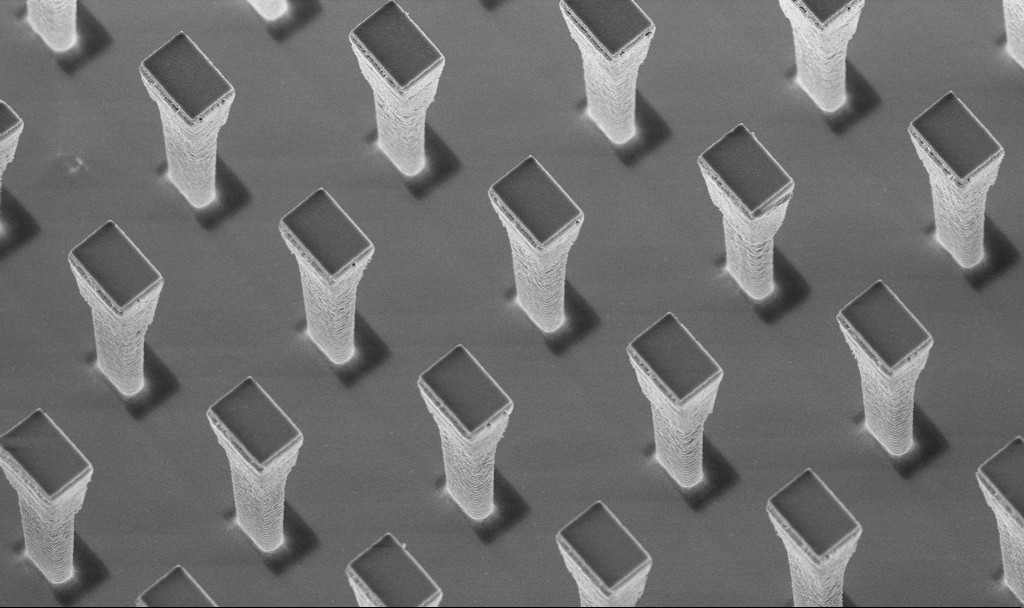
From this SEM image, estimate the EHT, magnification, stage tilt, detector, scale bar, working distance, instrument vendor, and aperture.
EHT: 5 kV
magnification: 6.66 K X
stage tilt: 20°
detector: InLens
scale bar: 10000 nm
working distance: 4.3 mm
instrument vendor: Zeiss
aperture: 30 µm